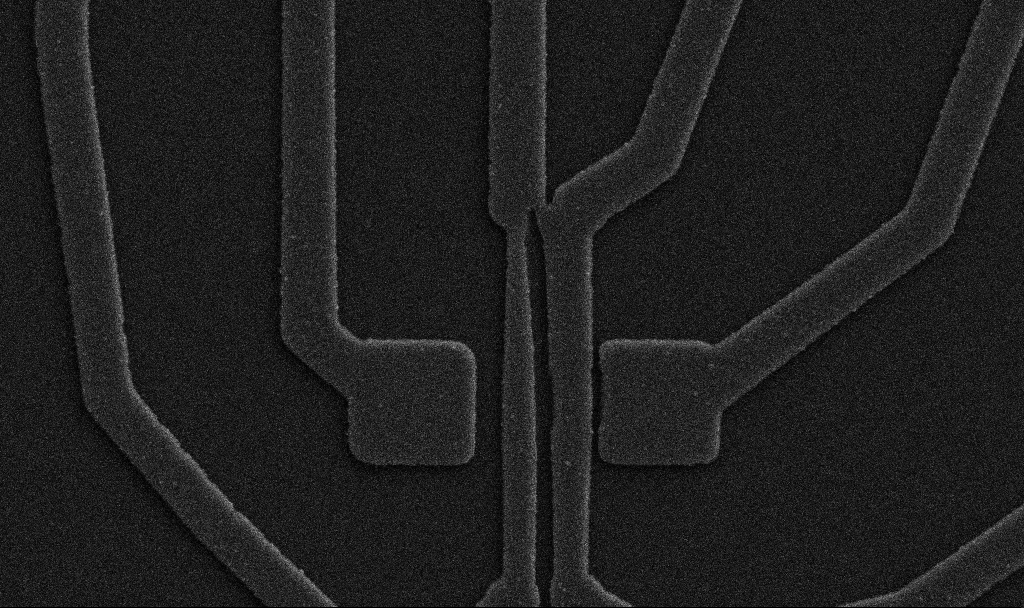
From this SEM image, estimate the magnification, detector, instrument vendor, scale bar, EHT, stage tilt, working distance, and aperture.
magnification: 18.34 K X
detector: SE2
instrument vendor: Zeiss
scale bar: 2000 nm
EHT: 5 kV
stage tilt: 0°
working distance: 10.7 mm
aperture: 30 µm